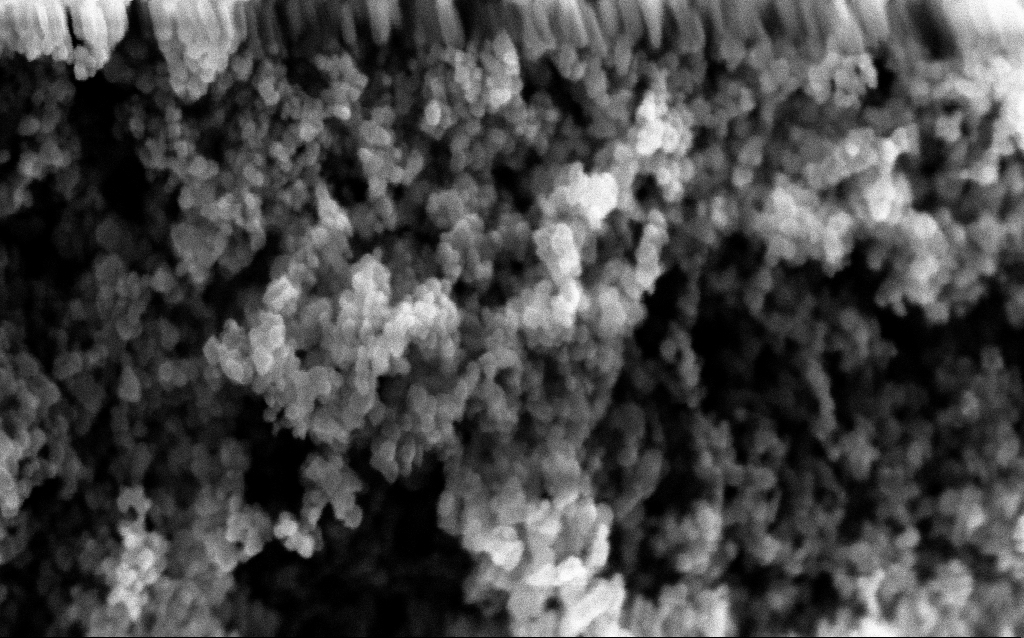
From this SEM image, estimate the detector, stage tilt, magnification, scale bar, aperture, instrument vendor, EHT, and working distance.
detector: InLens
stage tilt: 0°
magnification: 204.13 K X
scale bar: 100 nm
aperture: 30 µm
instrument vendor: Zeiss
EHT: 5 kV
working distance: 2.5 mm